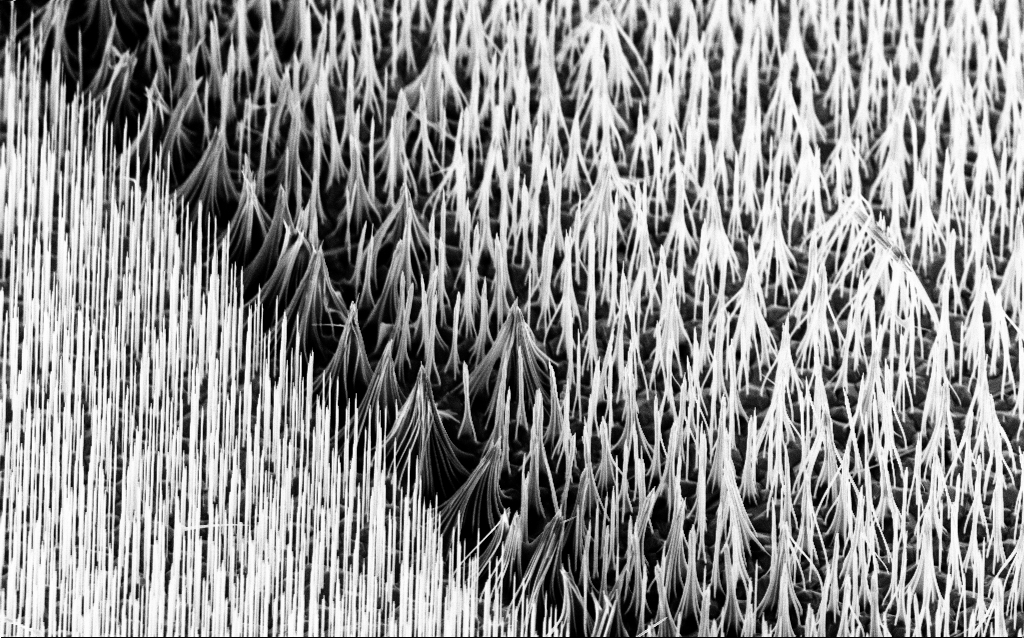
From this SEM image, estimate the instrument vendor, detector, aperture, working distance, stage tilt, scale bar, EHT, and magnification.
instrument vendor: Zeiss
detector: InLens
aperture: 30 µm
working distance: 6.2 mm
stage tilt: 40°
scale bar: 2000 nm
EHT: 5 kV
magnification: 10 K X